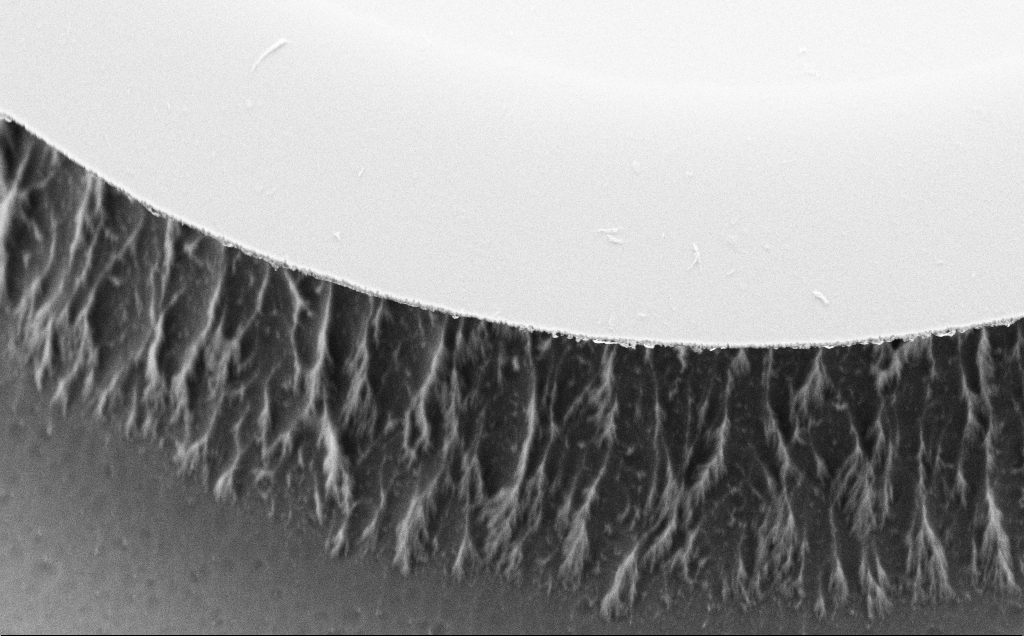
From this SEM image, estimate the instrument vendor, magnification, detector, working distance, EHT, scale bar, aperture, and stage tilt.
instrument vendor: Zeiss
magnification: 11.67 K X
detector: SE2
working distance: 7 mm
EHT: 5 kV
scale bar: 2000 nm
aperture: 30 µm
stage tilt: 45°